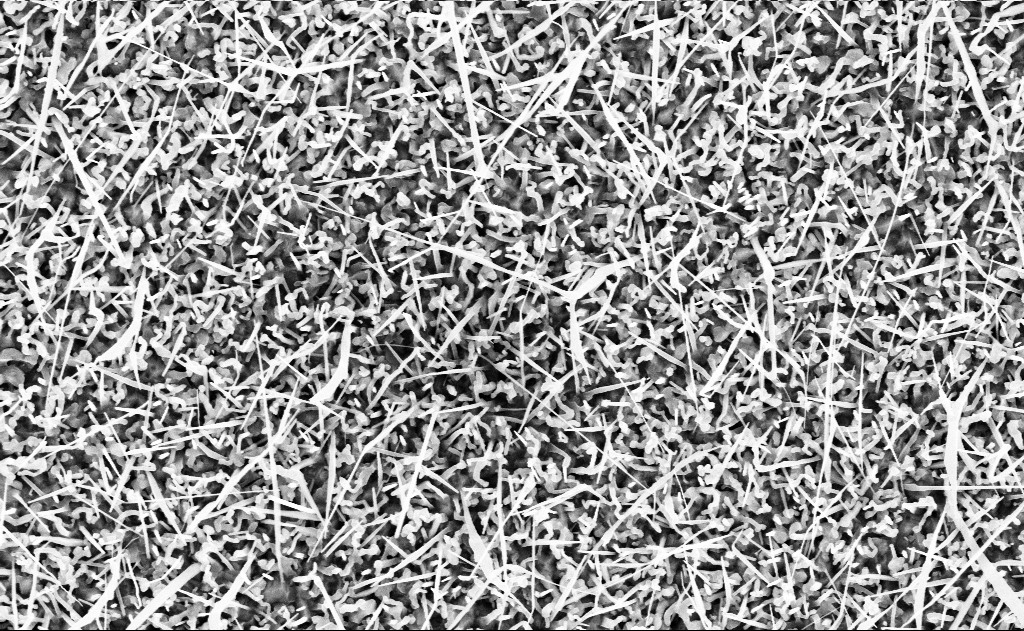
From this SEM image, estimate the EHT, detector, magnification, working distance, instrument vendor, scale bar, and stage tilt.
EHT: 10 kV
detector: InLens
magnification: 20 K X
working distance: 15 mm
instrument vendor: Zeiss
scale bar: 1000 nm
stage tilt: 0°